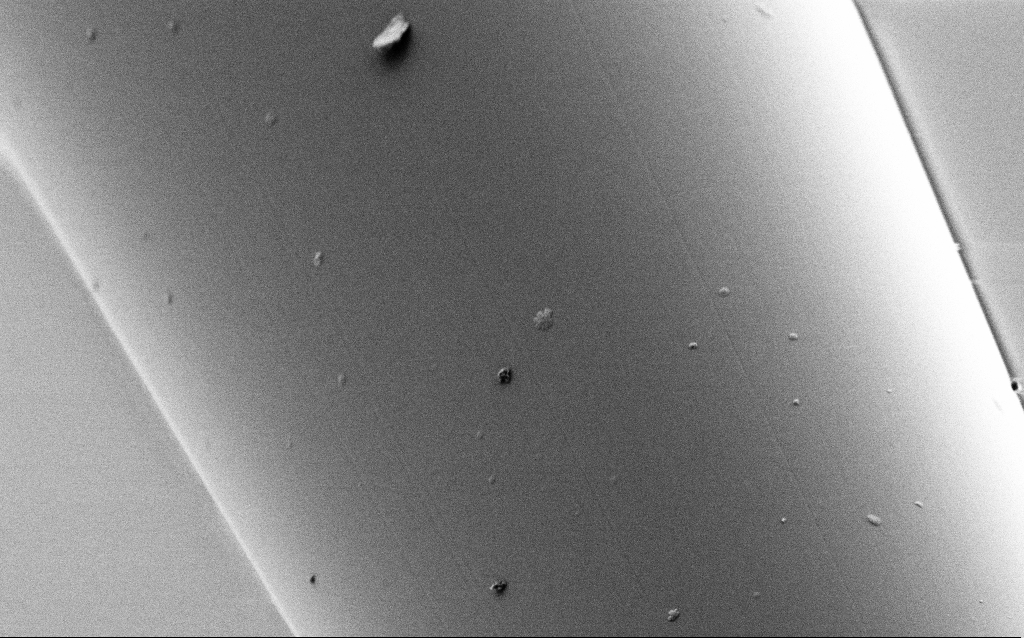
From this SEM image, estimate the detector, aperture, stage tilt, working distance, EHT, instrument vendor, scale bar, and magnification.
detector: SE2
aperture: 30 µm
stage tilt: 45°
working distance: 7.5 mm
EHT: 1 kV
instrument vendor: Zeiss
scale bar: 2000 nm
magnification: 20 K X